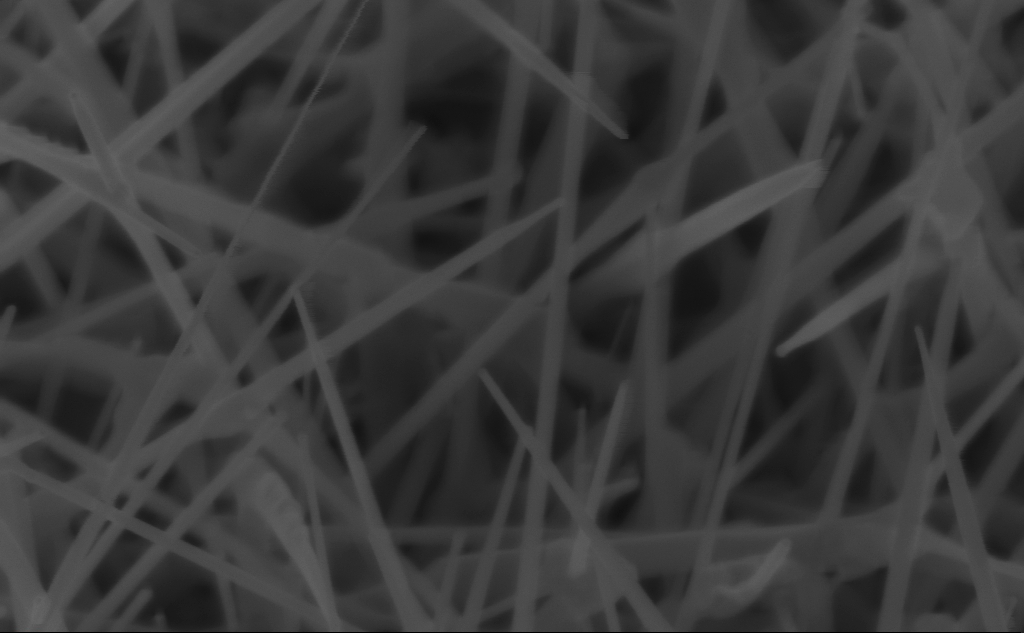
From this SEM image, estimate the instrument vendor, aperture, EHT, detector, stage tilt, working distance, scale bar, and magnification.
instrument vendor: Zeiss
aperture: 30 µm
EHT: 5 kV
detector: InLens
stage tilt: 45°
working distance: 4 mm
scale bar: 200 nm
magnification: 121.26 K X